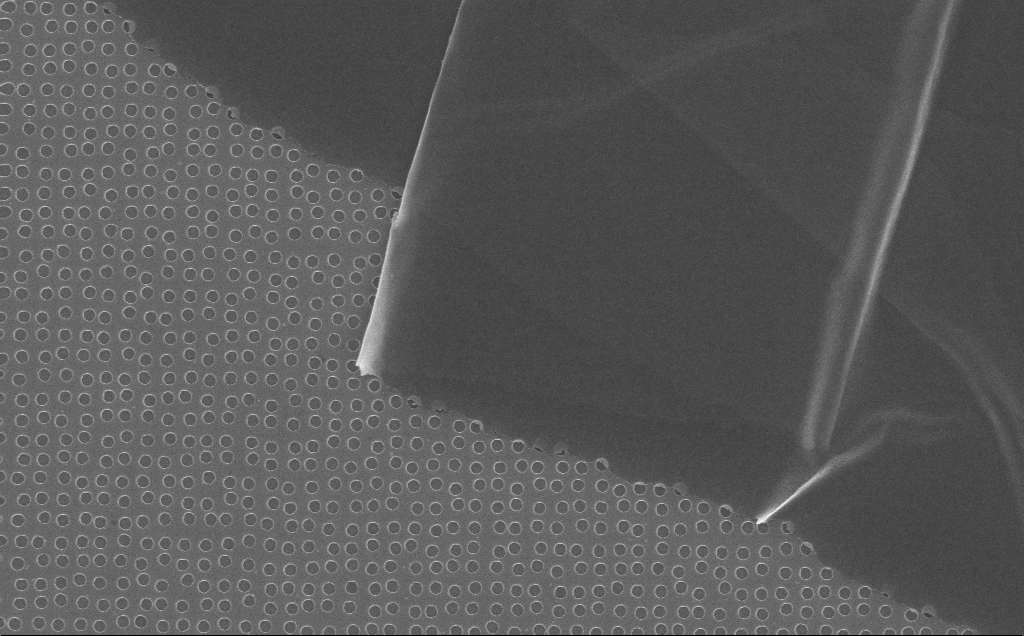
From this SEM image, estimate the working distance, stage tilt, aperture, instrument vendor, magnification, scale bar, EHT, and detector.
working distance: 7 mm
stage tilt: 0°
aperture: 30 µm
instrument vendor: Zeiss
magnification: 18.98 K X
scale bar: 2000 nm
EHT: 10 kV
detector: InLens